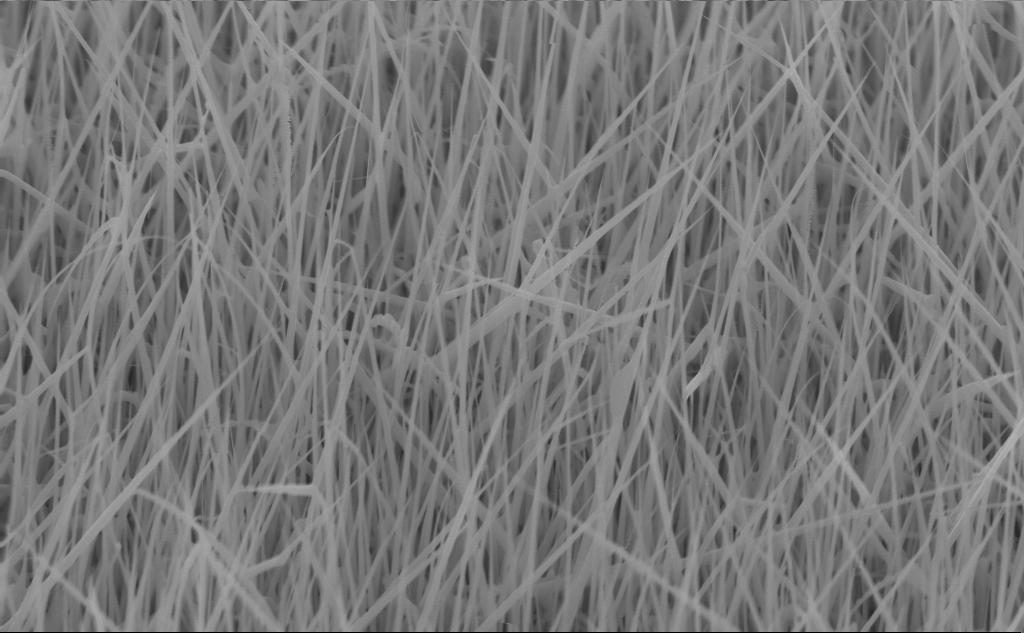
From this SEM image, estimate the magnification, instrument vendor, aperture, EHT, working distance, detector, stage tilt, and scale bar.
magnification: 40 K X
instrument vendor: Zeiss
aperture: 30 µm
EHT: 10 kV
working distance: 4 mm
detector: InLens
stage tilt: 45°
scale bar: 1000 nm